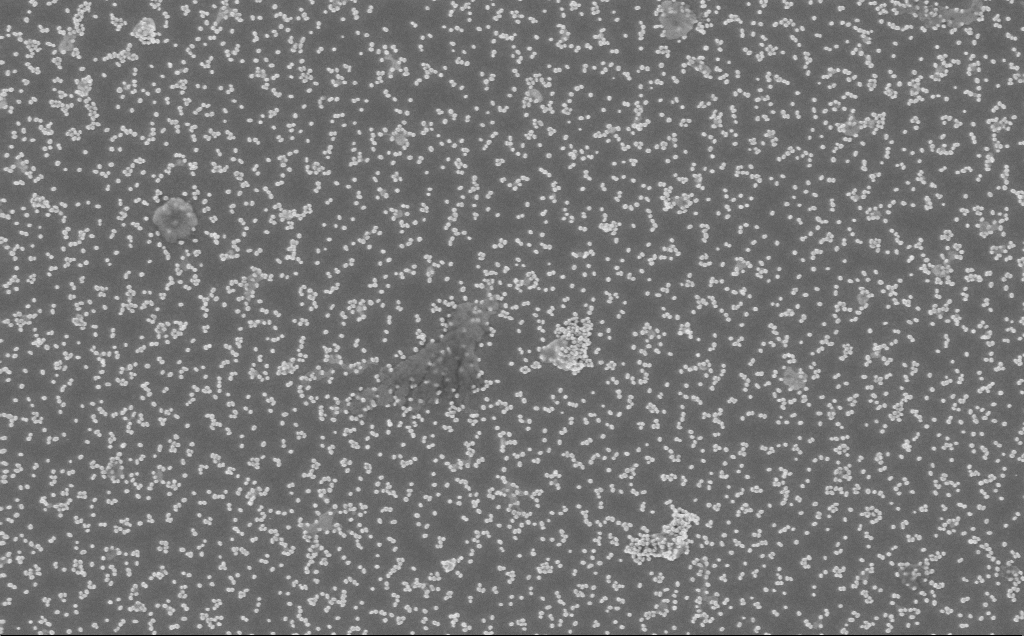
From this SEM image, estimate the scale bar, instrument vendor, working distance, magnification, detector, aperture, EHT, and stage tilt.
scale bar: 1000 nm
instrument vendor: Zeiss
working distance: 5 mm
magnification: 40 K X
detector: InLens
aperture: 30 µm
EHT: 3 kV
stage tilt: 0°